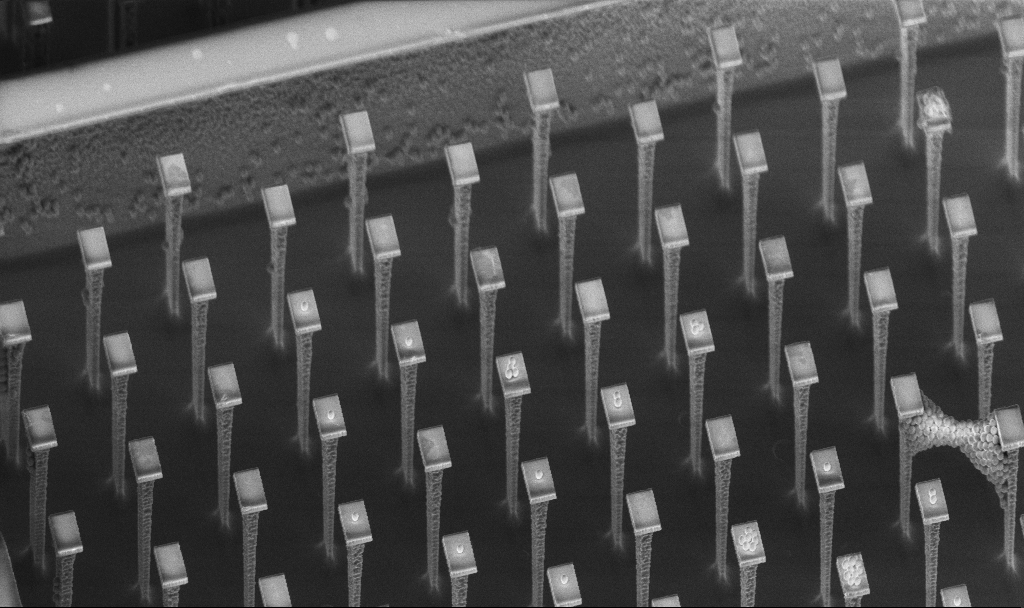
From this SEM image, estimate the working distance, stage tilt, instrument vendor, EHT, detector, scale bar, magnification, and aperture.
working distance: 4.3 mm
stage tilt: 45°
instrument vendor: Zeiss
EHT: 5 kV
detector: InLens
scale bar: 10000 nm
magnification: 3.34 K X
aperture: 30 µm